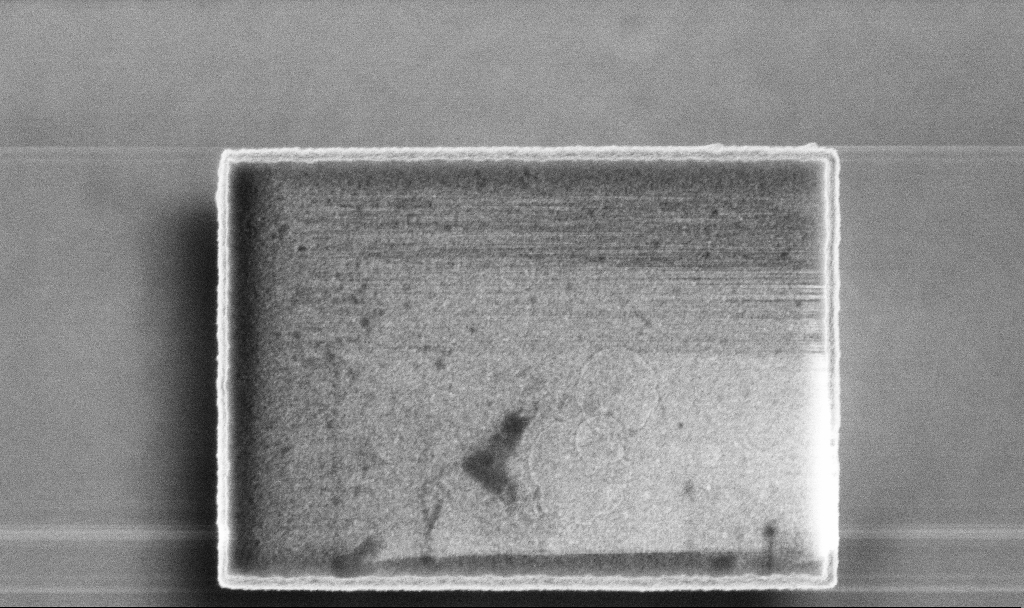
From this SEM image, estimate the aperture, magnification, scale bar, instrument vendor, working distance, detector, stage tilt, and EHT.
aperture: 30 µm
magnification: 50.15 K X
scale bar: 1000 nm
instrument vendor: Zeiss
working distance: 3.3 mm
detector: InLens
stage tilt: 0°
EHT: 5 kV